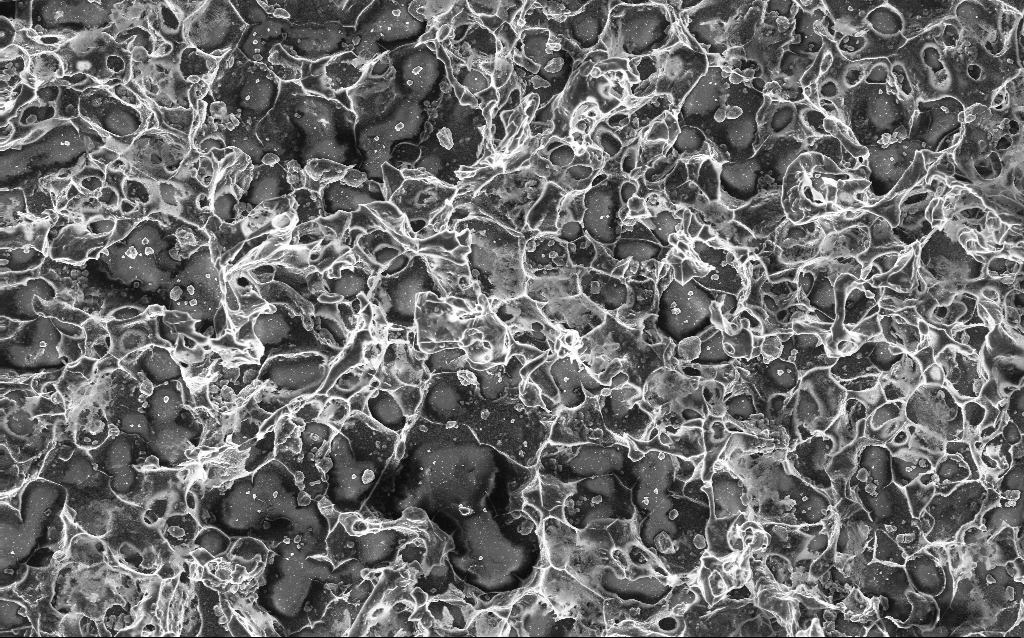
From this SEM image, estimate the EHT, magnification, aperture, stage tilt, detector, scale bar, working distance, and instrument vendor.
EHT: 10 kV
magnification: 0.792 K X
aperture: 30 µm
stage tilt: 0°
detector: InLens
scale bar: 20000 nm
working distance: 2.7 mm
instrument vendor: Zeiss